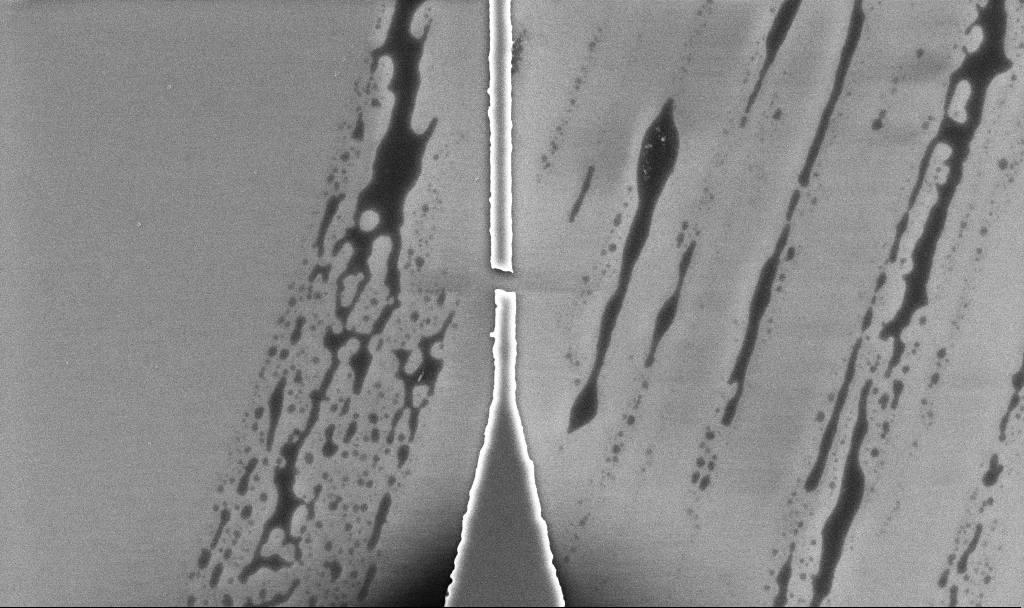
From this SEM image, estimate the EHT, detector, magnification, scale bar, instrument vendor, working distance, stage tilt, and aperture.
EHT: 5 kV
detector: InLens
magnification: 15.72 K X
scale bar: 2000 nm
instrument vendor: Zeiss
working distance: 5.2 mm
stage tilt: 0°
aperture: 30 µm